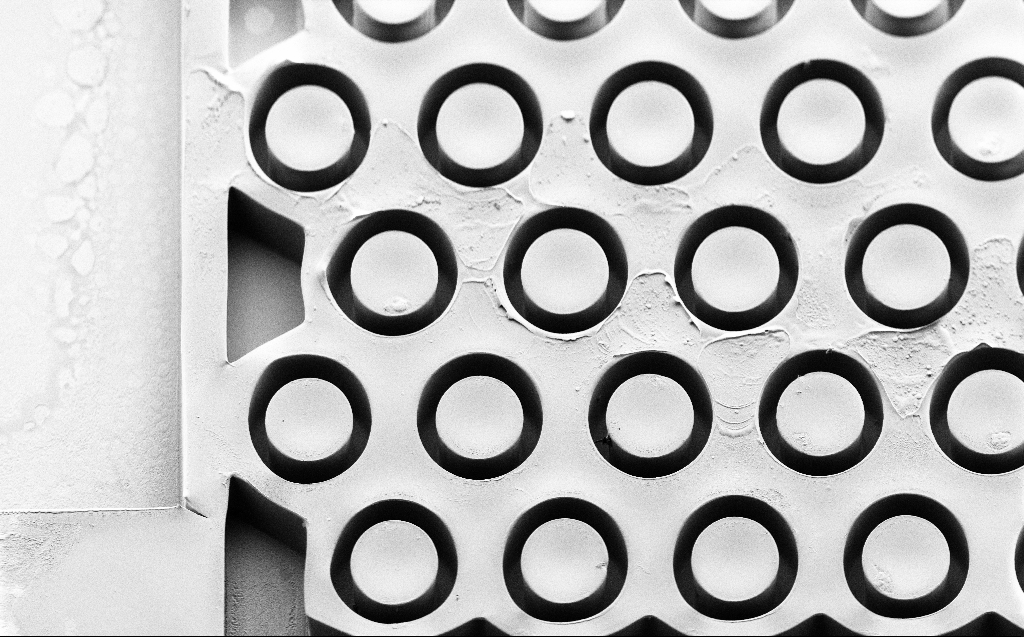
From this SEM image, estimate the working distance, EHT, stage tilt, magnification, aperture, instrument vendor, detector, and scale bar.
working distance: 7 mm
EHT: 2 kV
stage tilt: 45°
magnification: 0.785 K X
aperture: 30 µm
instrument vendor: Zeiss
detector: SE2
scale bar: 20000 nm